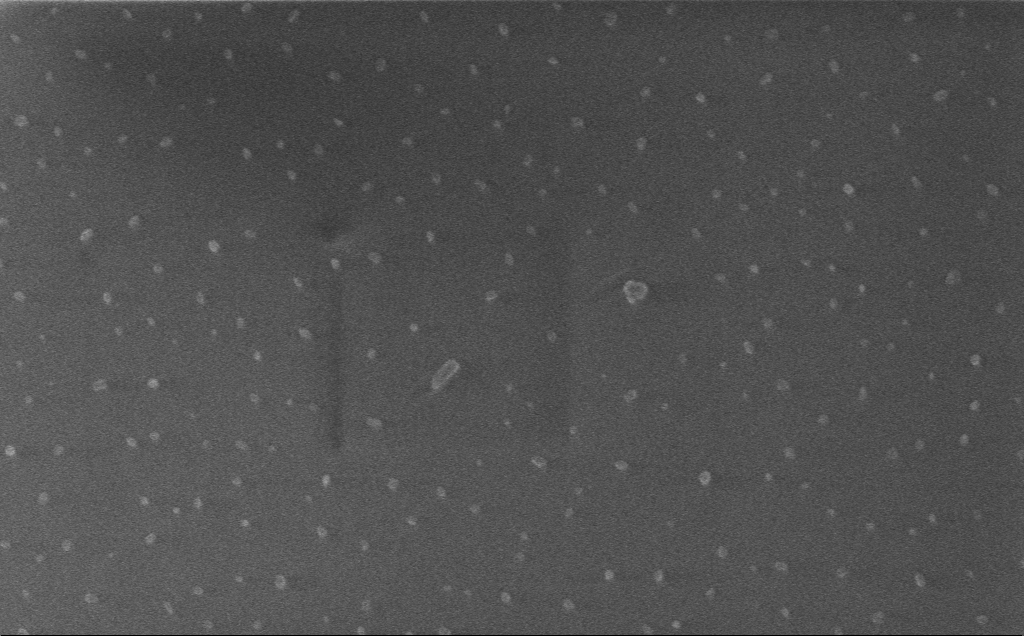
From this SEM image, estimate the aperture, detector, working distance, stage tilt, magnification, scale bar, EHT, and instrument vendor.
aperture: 30 µm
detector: InLens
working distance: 3 mm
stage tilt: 0°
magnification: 52.2 K X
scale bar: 1000 nm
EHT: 1 kV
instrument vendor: Zeiss